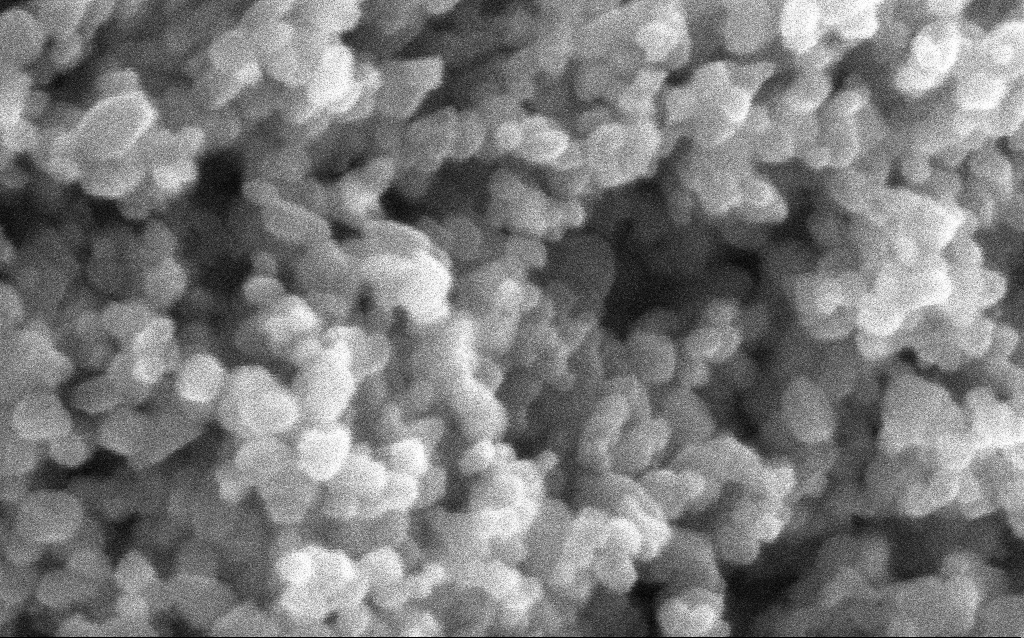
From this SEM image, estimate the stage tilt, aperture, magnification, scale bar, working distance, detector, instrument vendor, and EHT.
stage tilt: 0°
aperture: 30 µm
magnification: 600 K X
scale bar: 100 nm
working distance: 2.9 mm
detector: InLens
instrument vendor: Zeiss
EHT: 5 kV